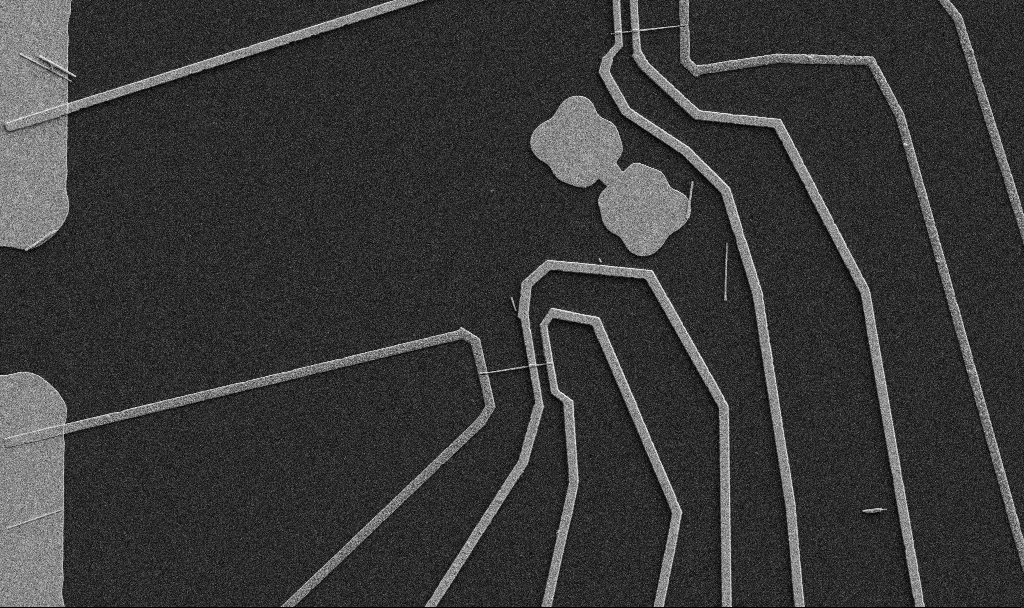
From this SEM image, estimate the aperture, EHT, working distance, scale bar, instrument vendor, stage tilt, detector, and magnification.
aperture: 30 µm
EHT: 5 kV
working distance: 10.7 mm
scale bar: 10000 nm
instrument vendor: Zeiss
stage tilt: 0°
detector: SE2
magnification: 5 K X